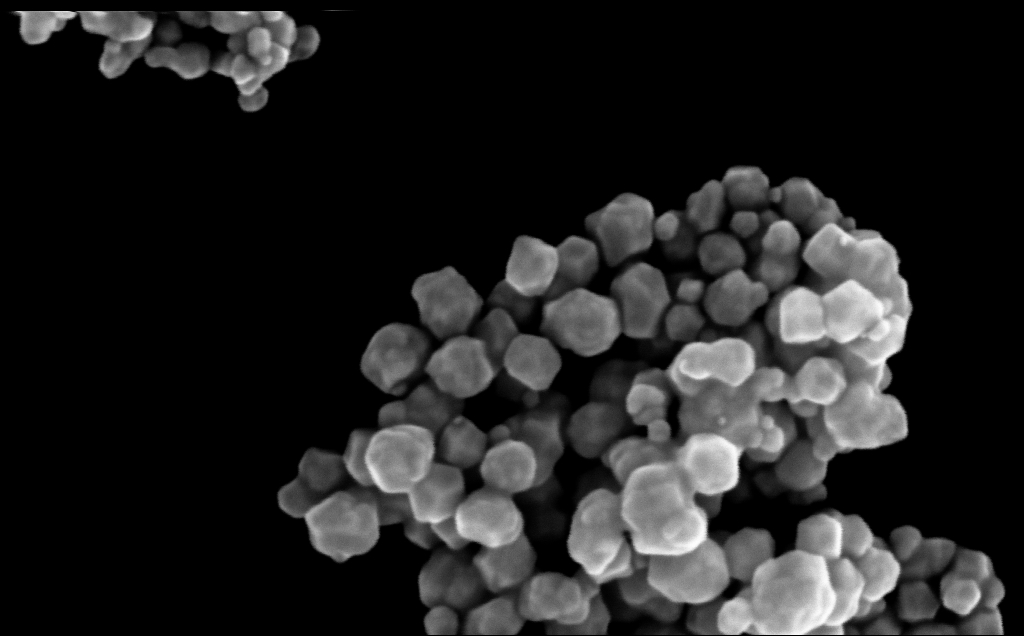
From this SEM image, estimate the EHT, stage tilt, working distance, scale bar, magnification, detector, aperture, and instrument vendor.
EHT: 10 kV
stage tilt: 0°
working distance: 3 mm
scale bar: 200 nm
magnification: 295.03 K X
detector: InLens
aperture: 30 µm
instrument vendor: Zeiss